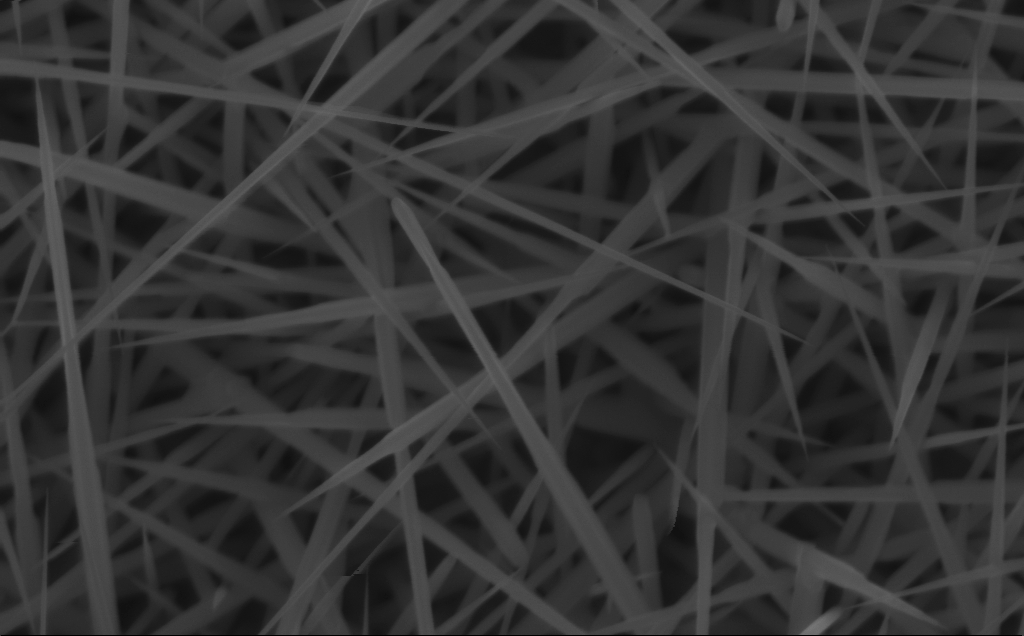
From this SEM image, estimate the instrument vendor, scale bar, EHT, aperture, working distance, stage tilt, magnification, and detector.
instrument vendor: Zeiss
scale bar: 200 nm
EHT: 10 kV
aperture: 30 µm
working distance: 4 mm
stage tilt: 0°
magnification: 80 K X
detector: InLens